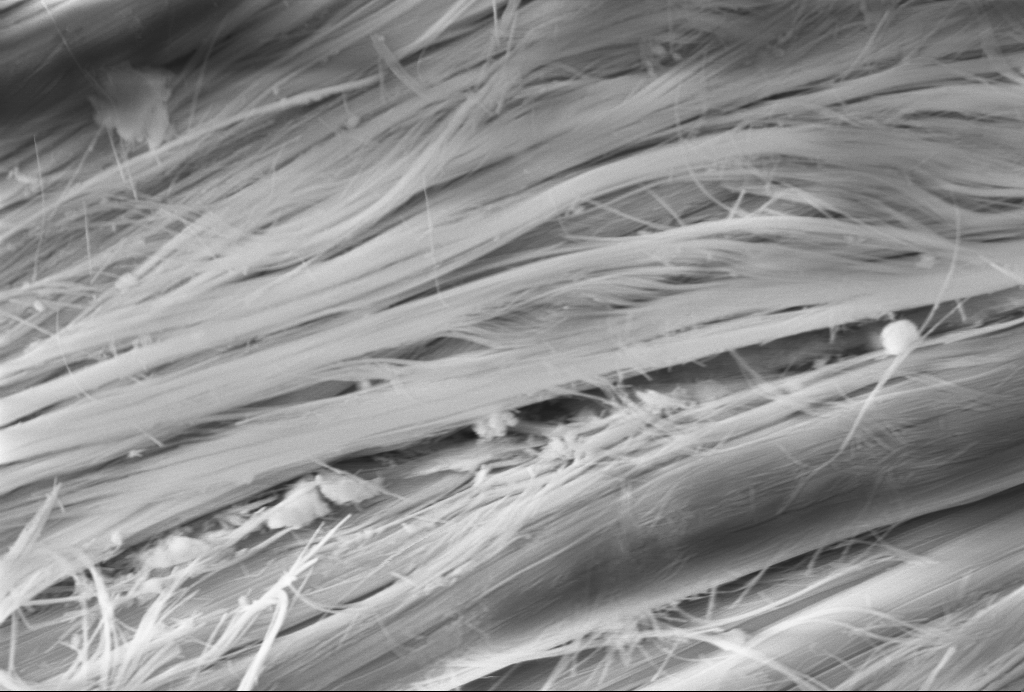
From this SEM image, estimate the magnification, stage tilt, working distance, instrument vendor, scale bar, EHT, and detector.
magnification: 17.21 K X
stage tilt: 45°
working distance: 7.7 mm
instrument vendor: Zeiss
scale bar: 1000 nm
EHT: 10 kV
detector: InLens